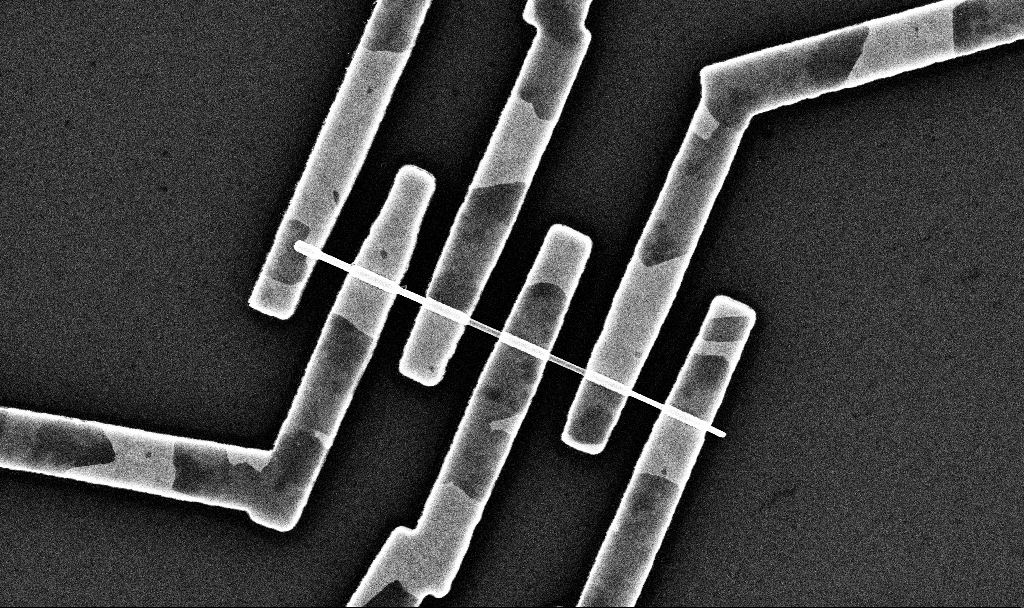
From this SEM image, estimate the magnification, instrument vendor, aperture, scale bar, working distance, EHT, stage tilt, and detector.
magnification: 27.72 K X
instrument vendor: Zeiss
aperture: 30 µm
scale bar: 2000 nm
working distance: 6.8 mm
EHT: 10 kV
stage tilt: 0°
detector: InLens